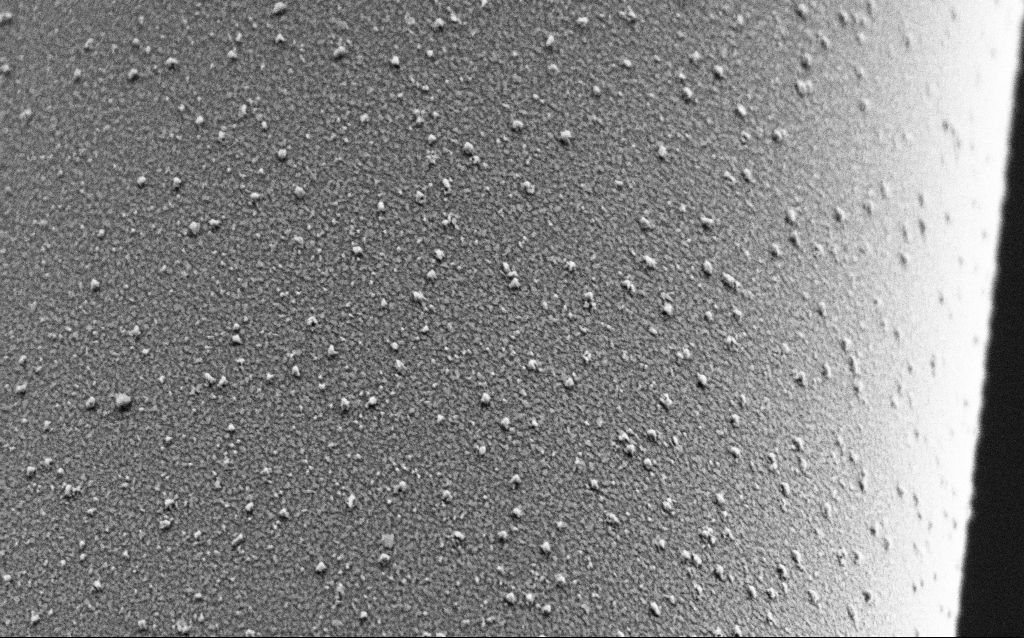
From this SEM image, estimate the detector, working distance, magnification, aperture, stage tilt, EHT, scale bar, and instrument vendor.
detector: SE2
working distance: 6.4 mm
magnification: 40 K X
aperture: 30 µm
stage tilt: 44.9°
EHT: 2 kV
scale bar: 1000 nm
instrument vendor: Zeiss